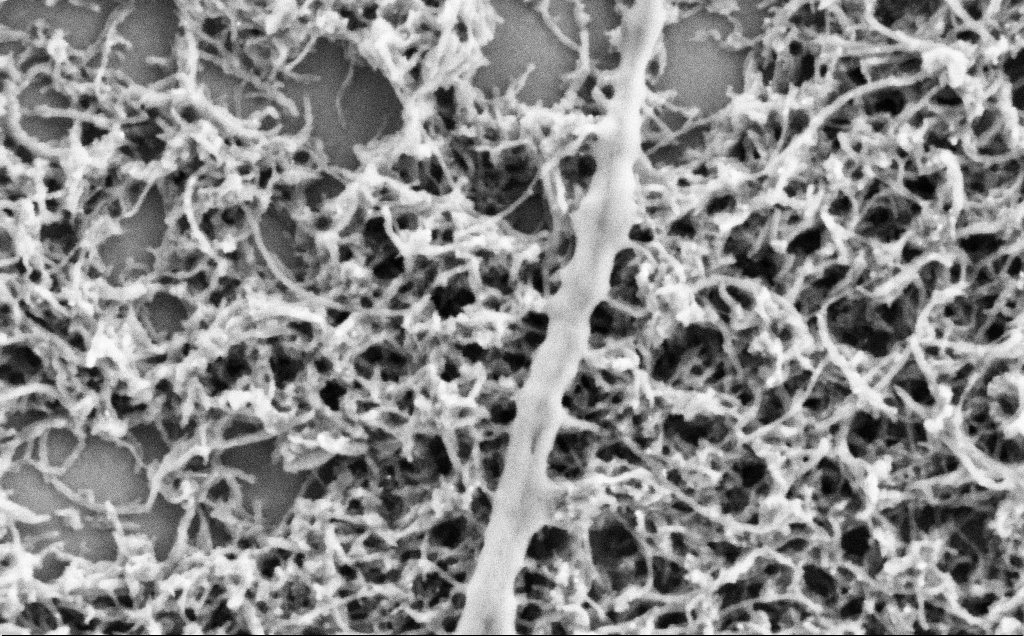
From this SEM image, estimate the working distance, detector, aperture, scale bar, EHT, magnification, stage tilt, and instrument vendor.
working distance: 7.1 mm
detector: SE2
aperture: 30 µm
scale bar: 200 nm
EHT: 2 kV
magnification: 80 K X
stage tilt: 0°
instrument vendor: Zeiss